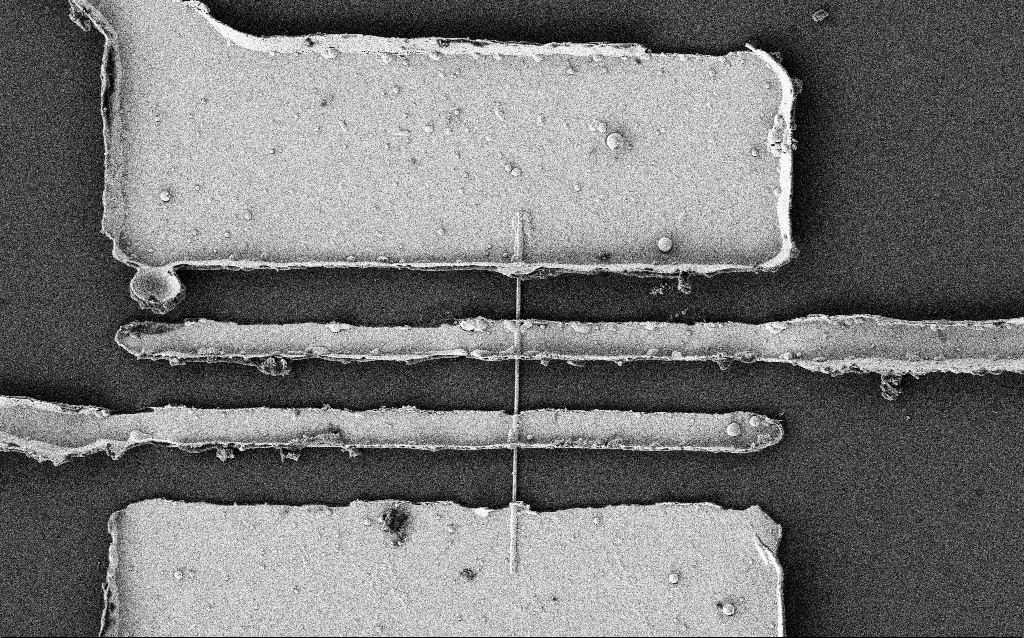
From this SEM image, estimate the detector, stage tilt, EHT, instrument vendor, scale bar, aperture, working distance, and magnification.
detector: SE2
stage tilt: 0°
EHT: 2 kV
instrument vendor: Zeiss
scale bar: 2000 nm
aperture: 30 µm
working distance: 7.4 mm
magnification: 8.21 K X